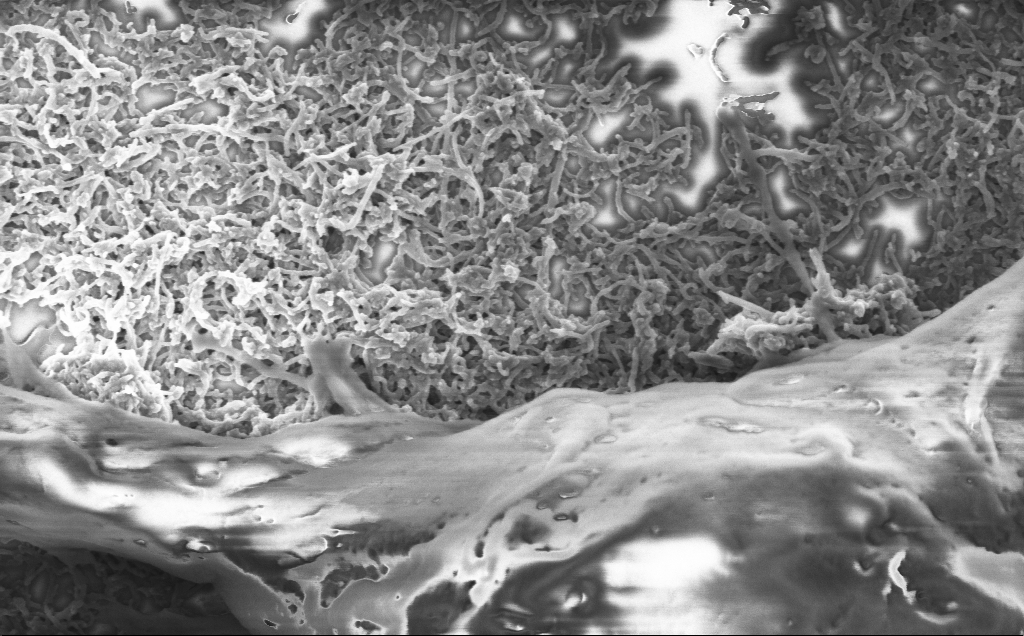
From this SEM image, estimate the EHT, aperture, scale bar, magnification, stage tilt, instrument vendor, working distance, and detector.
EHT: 2 kV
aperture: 30 µm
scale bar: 1000 nm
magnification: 50 K X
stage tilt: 0°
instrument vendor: Zeiss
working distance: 7.1 mm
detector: InLens